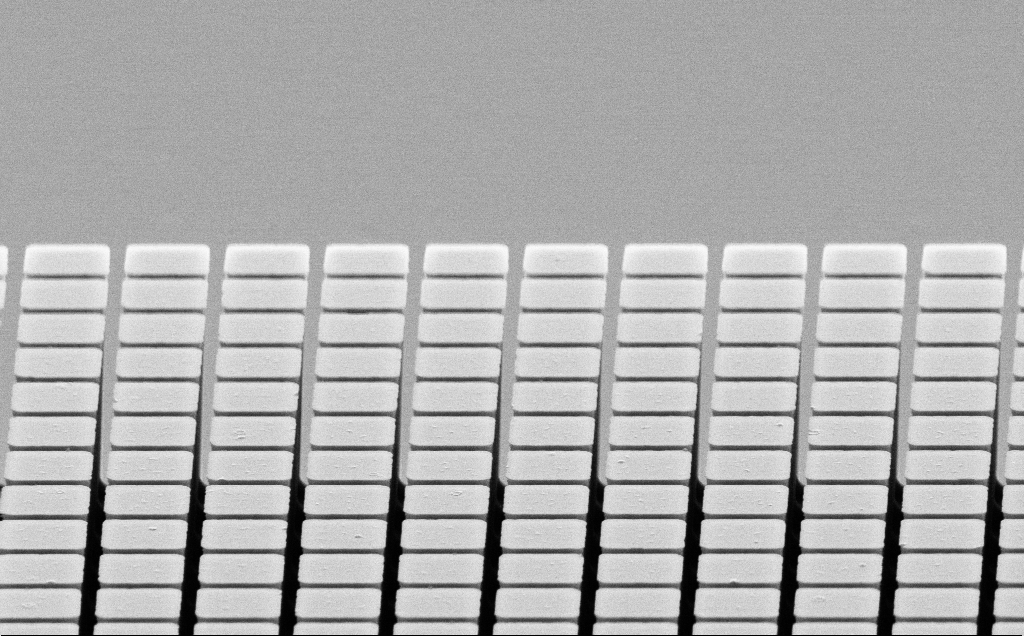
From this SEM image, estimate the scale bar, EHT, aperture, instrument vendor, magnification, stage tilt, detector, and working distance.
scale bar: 2000 nm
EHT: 10 kV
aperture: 30 µm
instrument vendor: Zeiss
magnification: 7.41 K X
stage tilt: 70°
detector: SE2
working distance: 7 mm